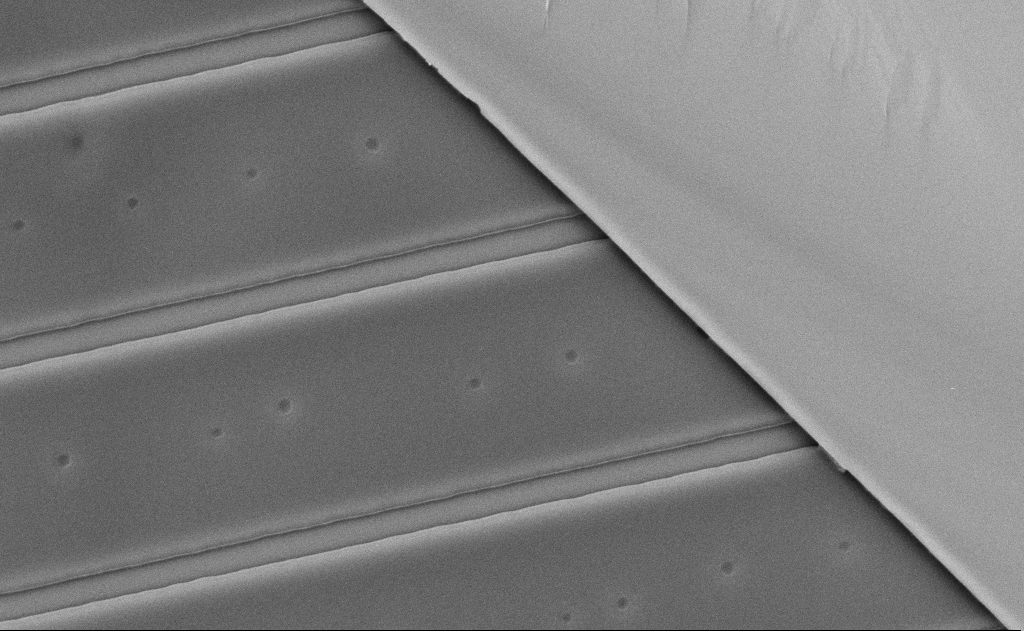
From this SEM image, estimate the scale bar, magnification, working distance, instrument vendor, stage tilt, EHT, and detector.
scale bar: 2000 nm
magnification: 22.74 K X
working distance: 12 mm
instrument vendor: Zeiss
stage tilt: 0°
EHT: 5 kV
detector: SE2